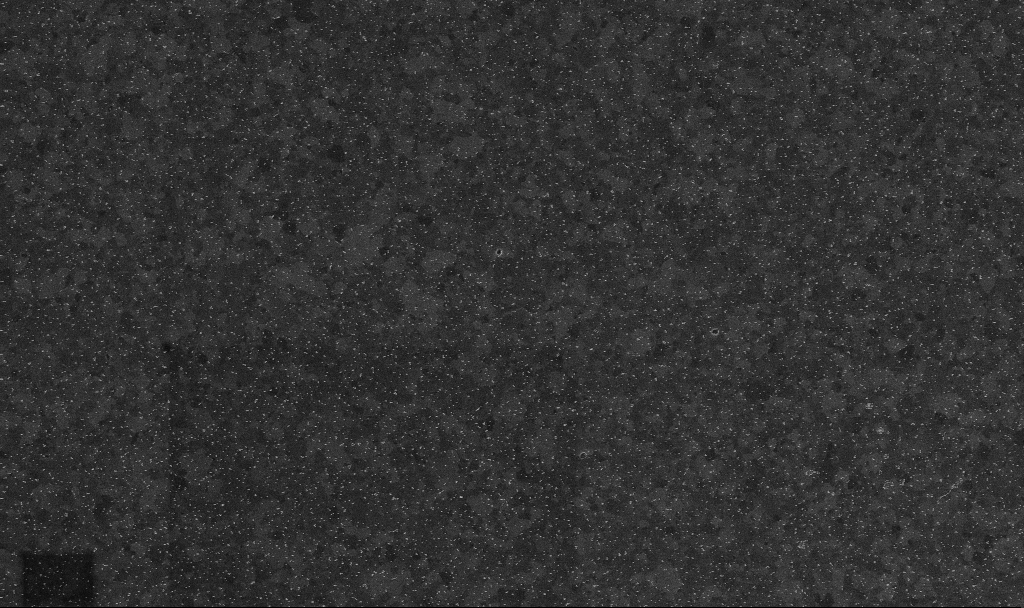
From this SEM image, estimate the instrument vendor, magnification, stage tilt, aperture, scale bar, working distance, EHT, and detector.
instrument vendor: Zeiss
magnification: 20 K X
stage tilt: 0°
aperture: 30 µm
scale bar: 1000 nm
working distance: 3.4 mm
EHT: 10 kV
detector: InLens